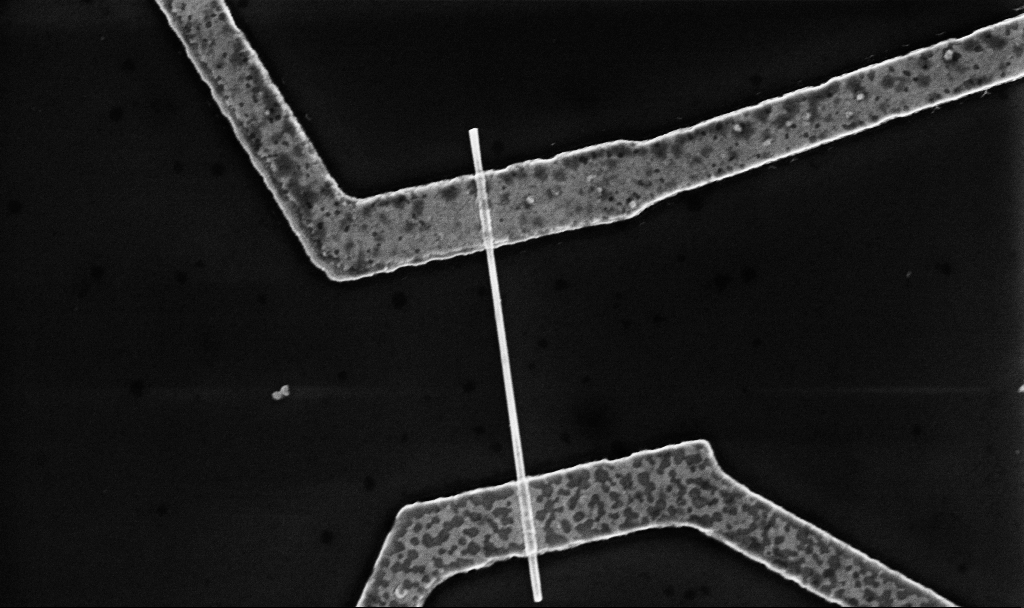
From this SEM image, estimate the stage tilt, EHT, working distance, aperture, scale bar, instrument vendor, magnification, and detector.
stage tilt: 0°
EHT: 5 kV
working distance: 8.7 mm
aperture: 30 µm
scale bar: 1000 nm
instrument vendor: Zeiss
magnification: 30 K X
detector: InLens